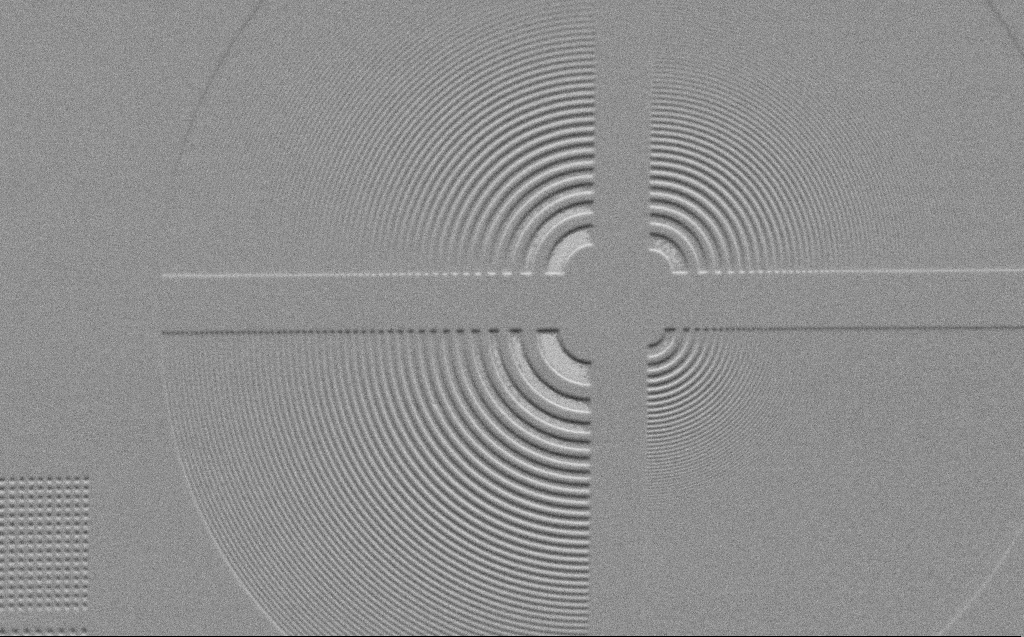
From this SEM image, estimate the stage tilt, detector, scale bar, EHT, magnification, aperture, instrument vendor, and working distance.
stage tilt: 45°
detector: SE2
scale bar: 10000 nm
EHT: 1 kV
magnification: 2.13 K X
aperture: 30 µm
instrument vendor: Zeiss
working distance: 5 mm